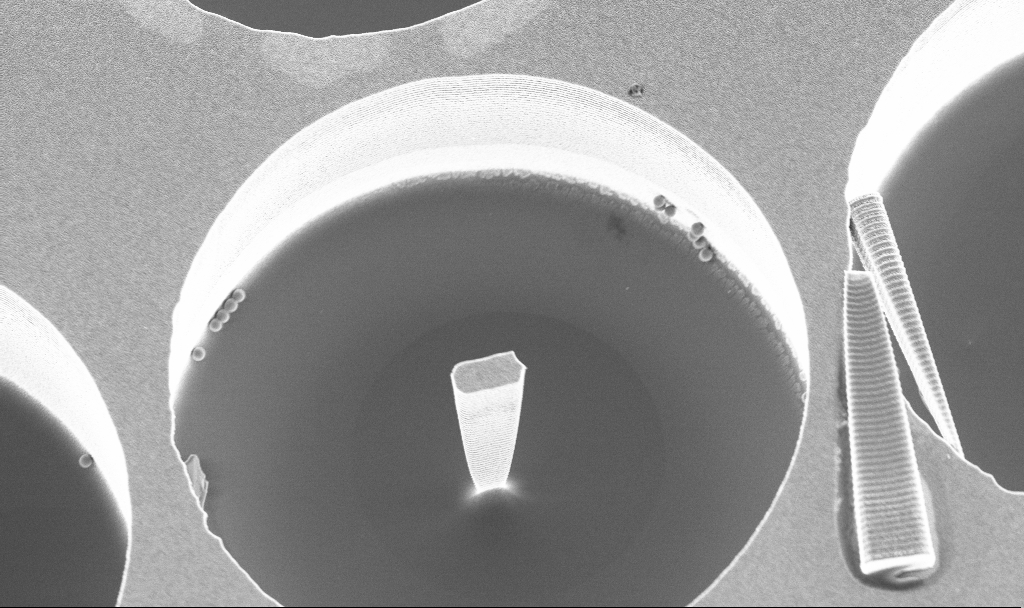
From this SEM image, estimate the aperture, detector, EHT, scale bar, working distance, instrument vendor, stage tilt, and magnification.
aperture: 30 µm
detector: InLens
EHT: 3 kV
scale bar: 2000 nm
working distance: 3.8 mm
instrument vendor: Zeiss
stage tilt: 20°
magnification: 7.77 K X